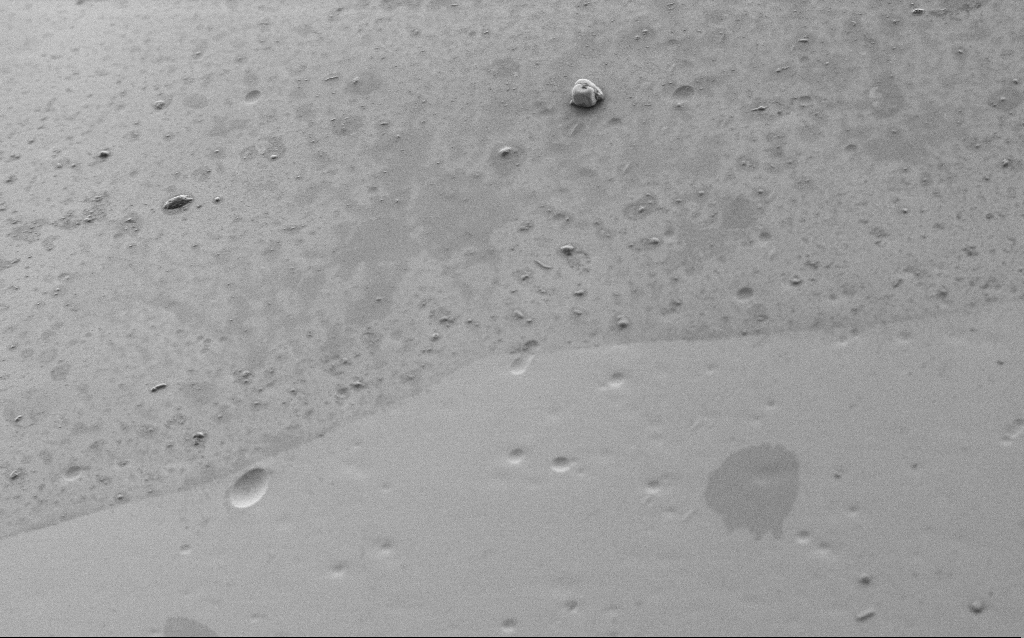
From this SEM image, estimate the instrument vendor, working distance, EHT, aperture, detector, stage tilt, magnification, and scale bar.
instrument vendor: Zeiss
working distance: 7.1 mm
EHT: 1 kV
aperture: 30 µm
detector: SE2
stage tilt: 45°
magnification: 2.5 K X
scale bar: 10000 nm